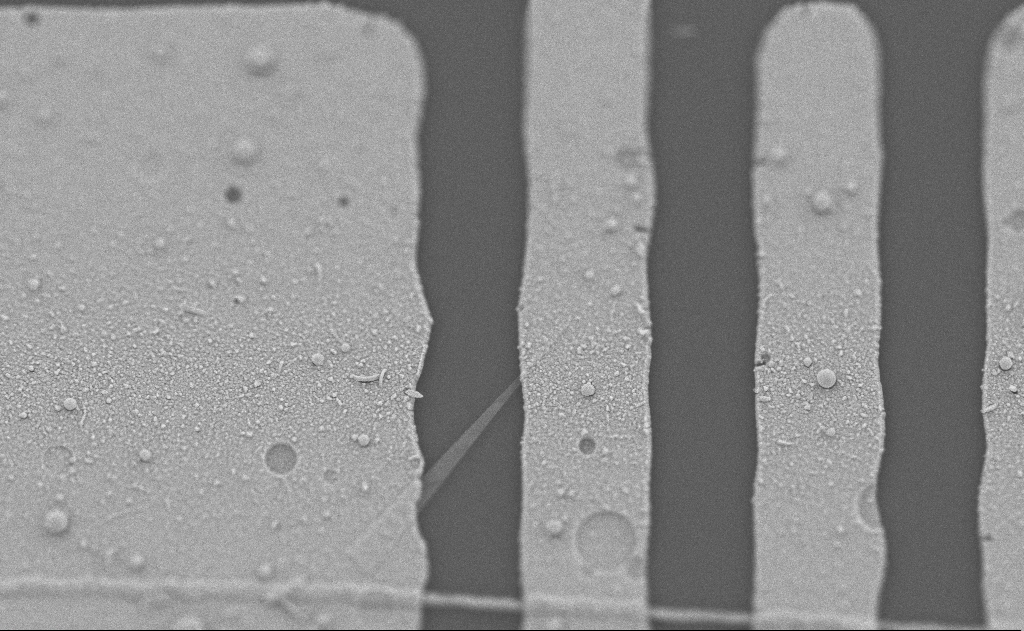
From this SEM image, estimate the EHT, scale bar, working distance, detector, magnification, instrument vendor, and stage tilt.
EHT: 5 kV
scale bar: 1000 nm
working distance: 10 mm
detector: SE2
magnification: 21.11 K X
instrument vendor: Zeiss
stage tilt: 0°